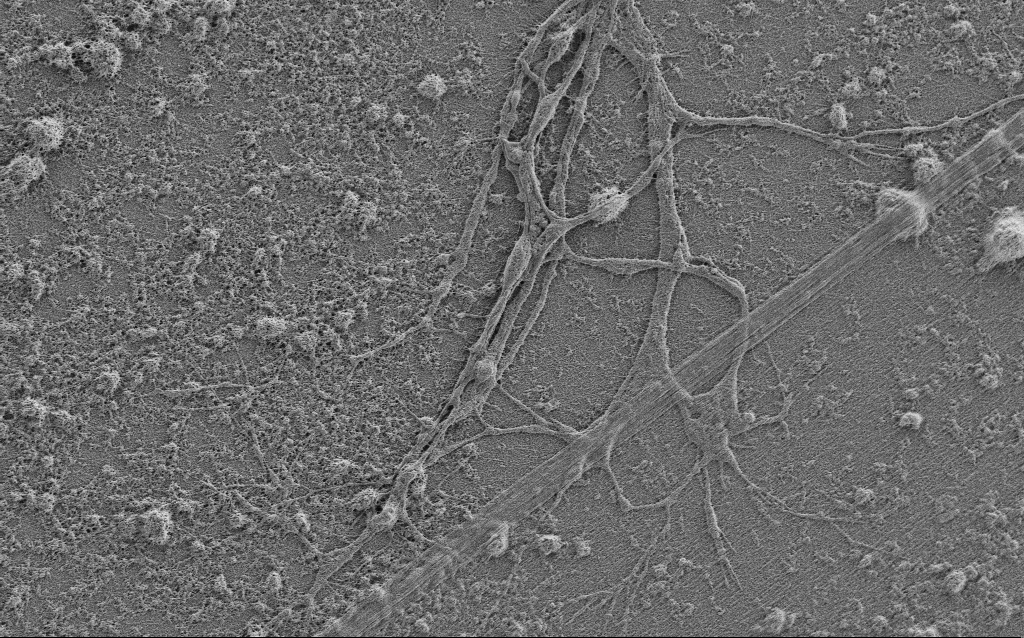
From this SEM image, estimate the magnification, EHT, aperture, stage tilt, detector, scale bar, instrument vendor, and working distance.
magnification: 5 K X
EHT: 0.9 kV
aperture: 30 µm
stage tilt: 0°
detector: SE2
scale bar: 10000 nm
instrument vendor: Zeiss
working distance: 4 mm